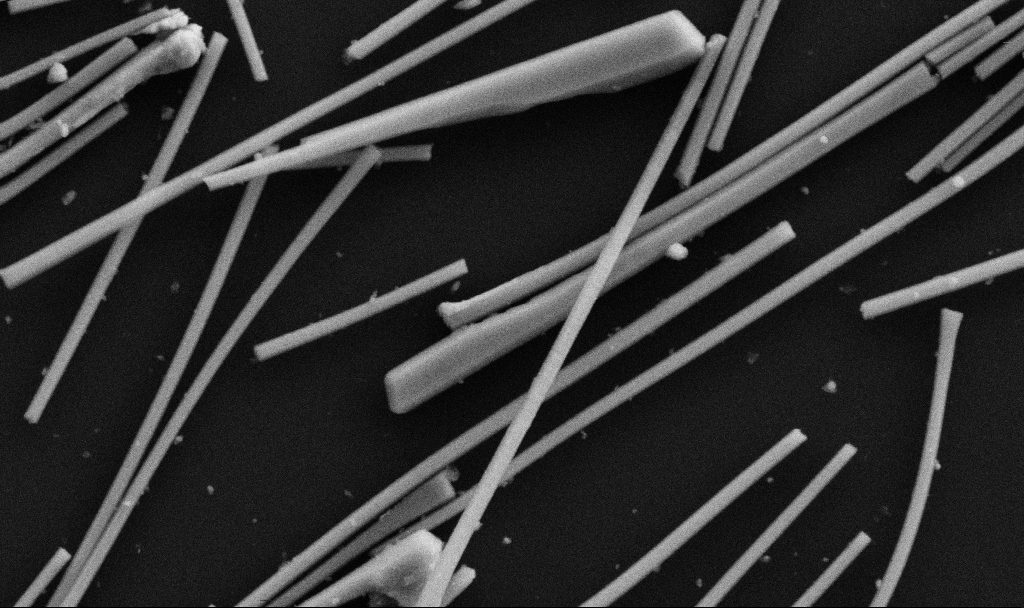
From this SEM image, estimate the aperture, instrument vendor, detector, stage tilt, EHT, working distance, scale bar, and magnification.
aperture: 30 µm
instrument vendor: Zeiss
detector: SE2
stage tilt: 0°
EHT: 5 kV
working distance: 6.7 mm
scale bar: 1000 nm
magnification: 63.2 K X